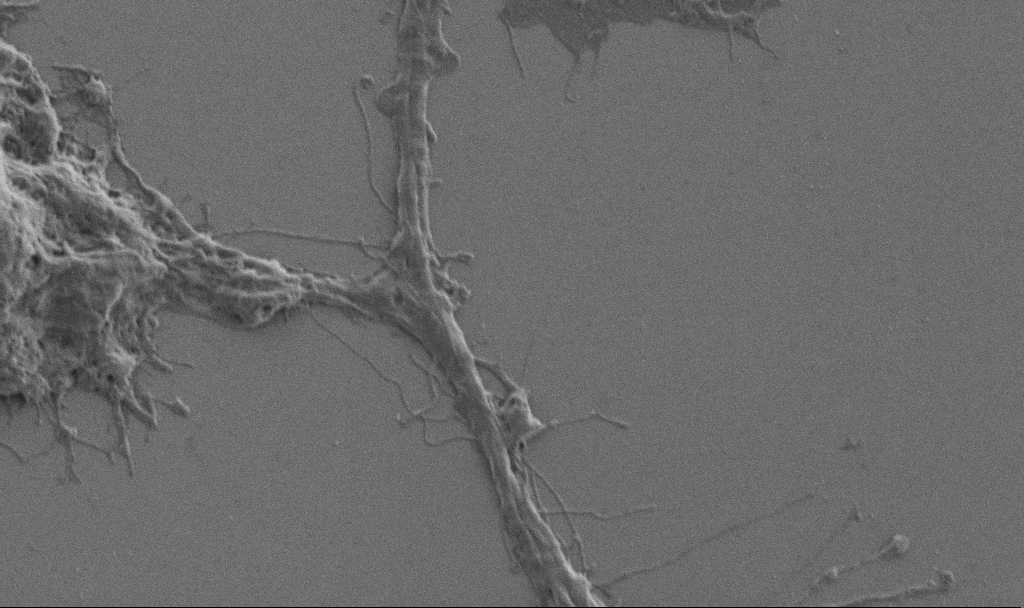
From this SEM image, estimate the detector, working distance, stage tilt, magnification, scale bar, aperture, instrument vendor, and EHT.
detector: SE2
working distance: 6.9 mm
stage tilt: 0°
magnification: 10 K X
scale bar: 2000 nm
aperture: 30 µm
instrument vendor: Zeiss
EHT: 1 kV